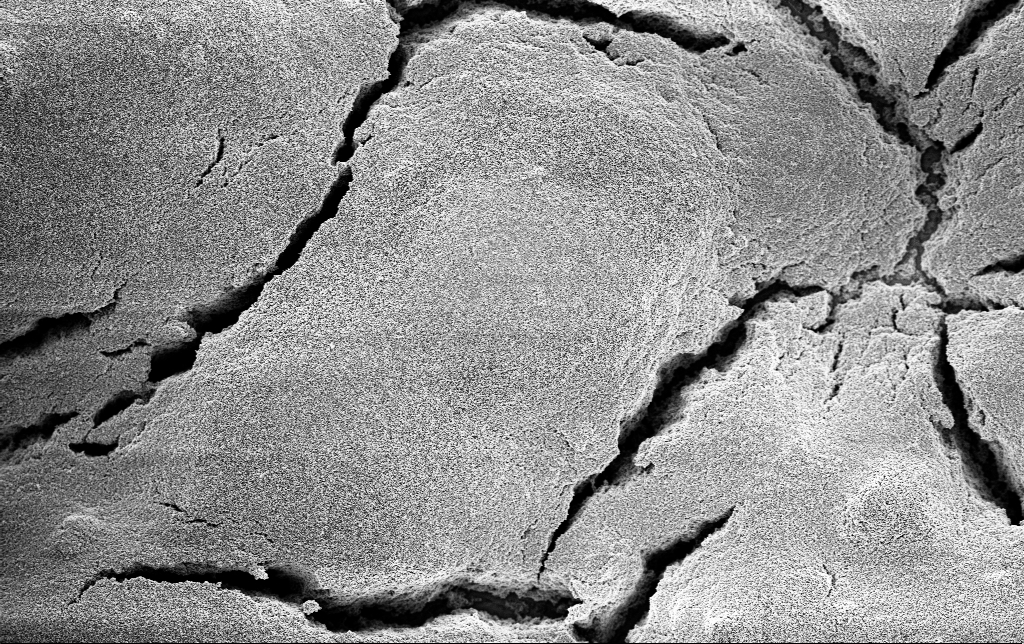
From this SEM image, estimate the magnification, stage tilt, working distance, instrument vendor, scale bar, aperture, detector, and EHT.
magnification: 3 K X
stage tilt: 0°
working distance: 2.8 mm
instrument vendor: Zeiss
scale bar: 10000 nm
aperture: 30 µm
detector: InLens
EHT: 3 kV